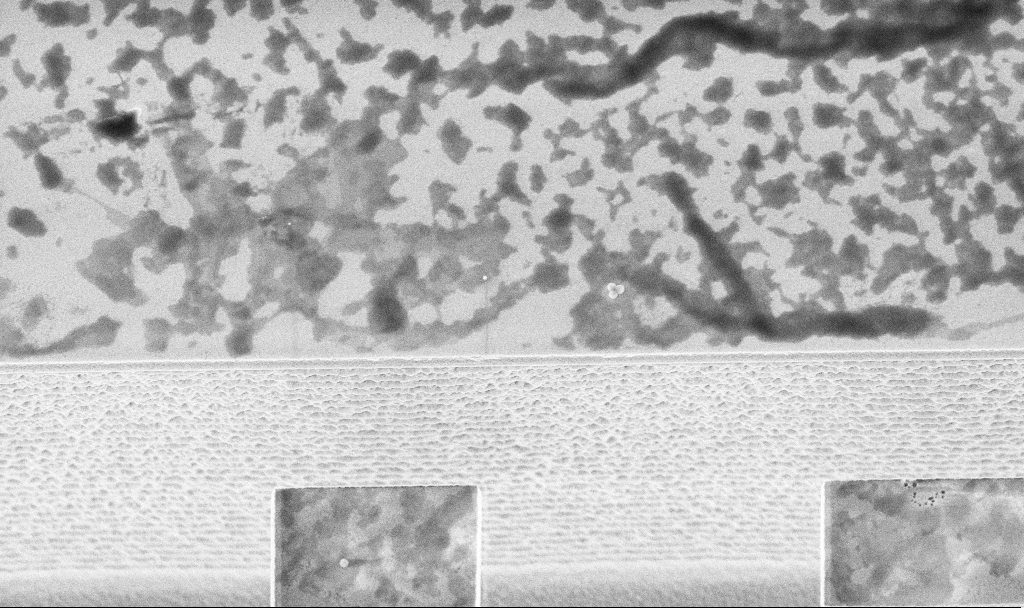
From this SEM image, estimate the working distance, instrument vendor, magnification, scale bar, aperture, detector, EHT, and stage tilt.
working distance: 4.8 mm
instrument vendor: Zeiss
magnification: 16.77 K X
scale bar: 1000 nm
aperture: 30 µm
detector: InLens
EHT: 3 kV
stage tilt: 20°